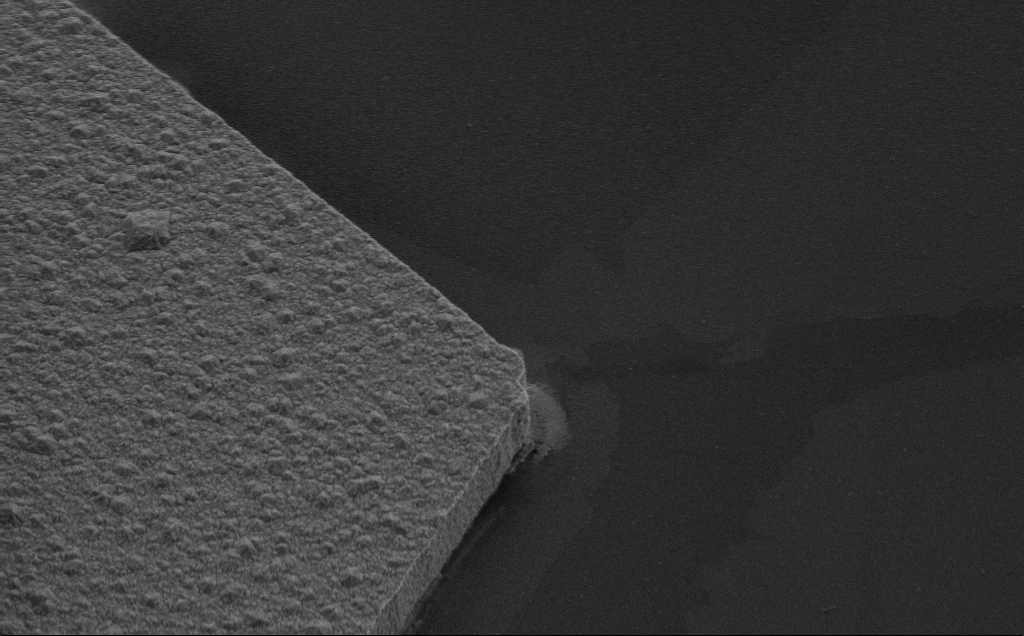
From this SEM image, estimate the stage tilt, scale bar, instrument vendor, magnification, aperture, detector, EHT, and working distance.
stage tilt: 35°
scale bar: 10000 nm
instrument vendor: Zeiss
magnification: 6.56 K X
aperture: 30 µm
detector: SE2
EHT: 10 kV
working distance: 8 mm